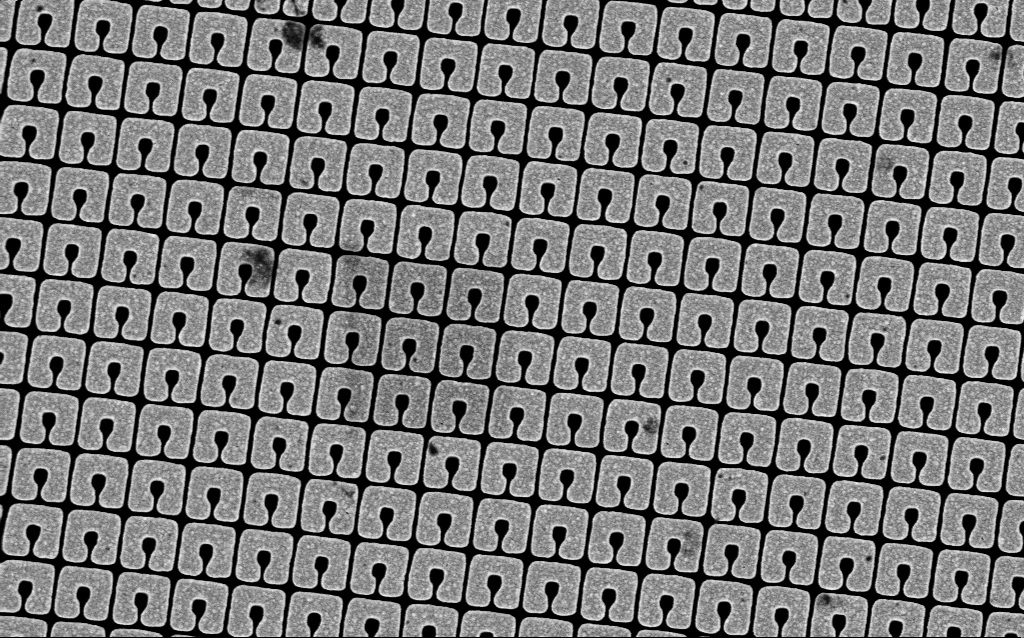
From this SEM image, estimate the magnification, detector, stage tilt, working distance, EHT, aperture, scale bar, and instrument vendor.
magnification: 46.08 K X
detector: InLens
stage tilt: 0°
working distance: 6.8 mm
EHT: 3 kV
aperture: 30 µm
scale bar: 1000 nm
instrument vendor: Zeiss